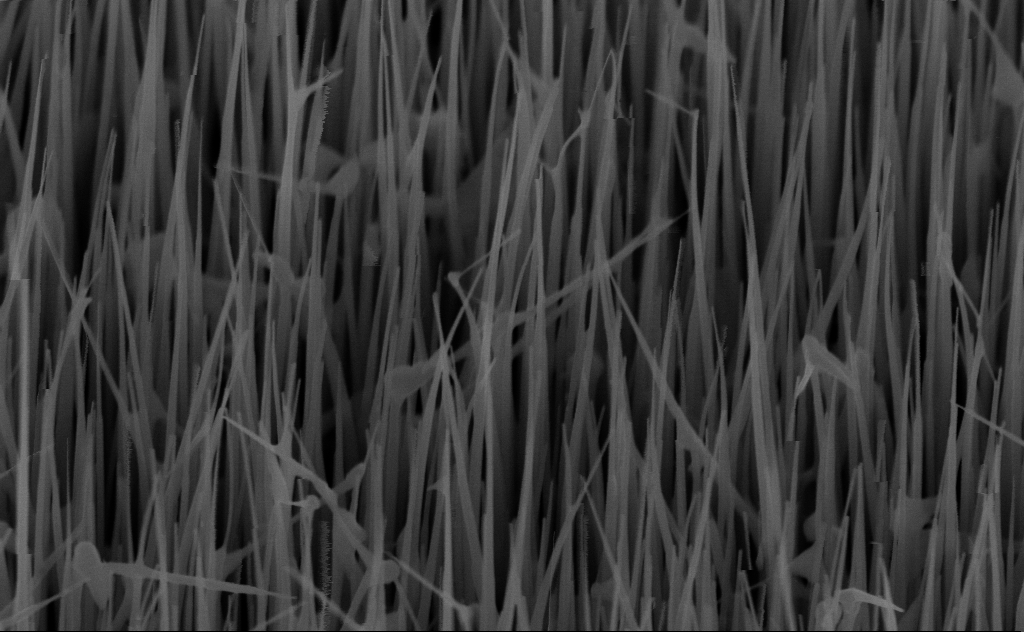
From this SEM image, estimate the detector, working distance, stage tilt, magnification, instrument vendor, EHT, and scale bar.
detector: InLens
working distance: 6 mm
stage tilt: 45°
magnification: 80 K X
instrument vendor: Zeiss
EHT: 10 kV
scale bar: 200 nm